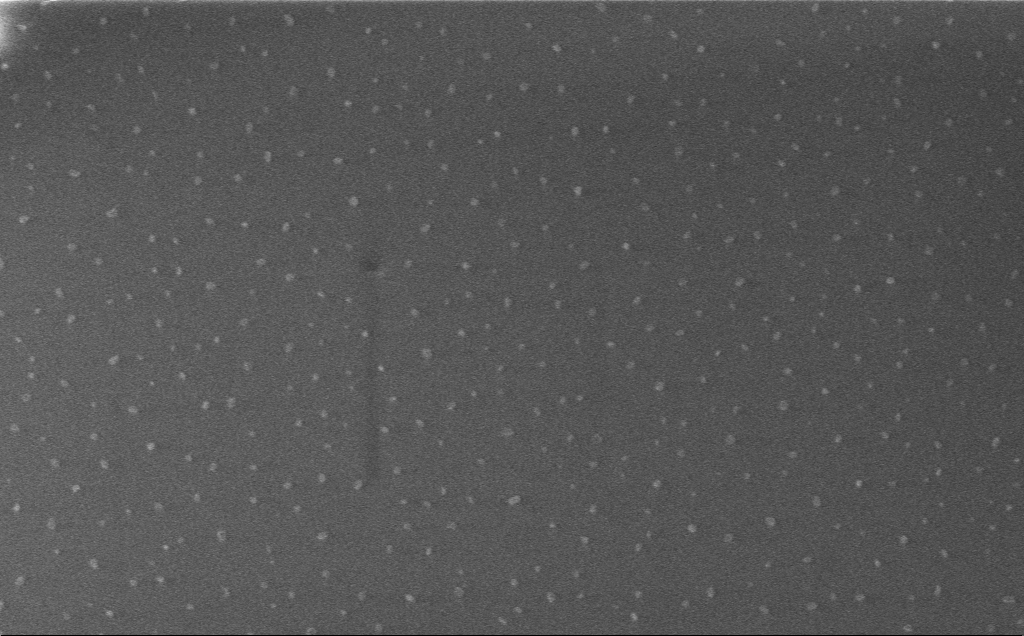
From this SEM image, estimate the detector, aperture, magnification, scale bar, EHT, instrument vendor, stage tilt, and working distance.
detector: InLens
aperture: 30 µm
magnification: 38.61 K X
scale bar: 1000 nm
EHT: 1 kV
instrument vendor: Zeiss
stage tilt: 0°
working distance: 3 mm